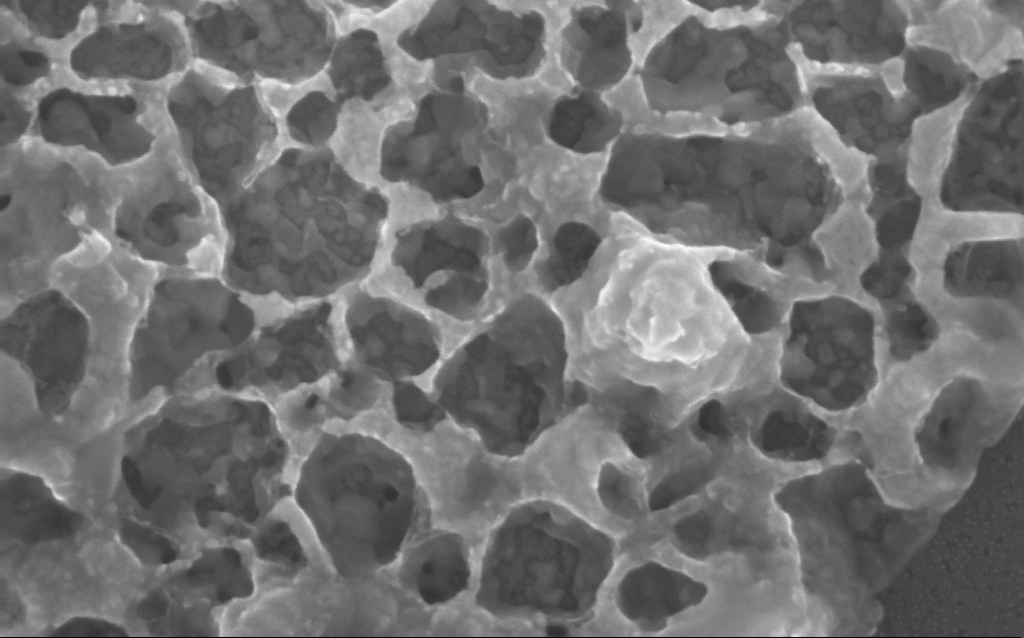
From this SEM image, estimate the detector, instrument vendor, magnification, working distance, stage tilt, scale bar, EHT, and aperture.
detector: InLens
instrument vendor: Zeiss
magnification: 310.66 K X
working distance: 2 mm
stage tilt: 0°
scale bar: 200 nm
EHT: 10 kV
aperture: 30 µm